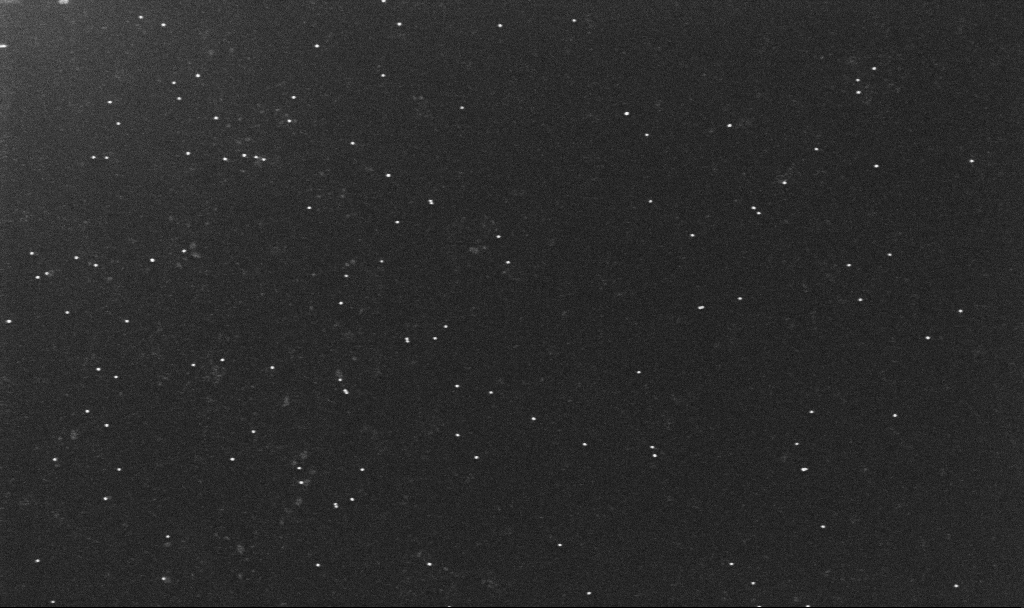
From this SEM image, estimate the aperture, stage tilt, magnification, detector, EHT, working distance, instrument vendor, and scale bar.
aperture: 30 µm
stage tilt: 0°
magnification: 100 K X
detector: InLens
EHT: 10 kV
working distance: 3.2 mm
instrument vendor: Zeiss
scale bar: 200 nm